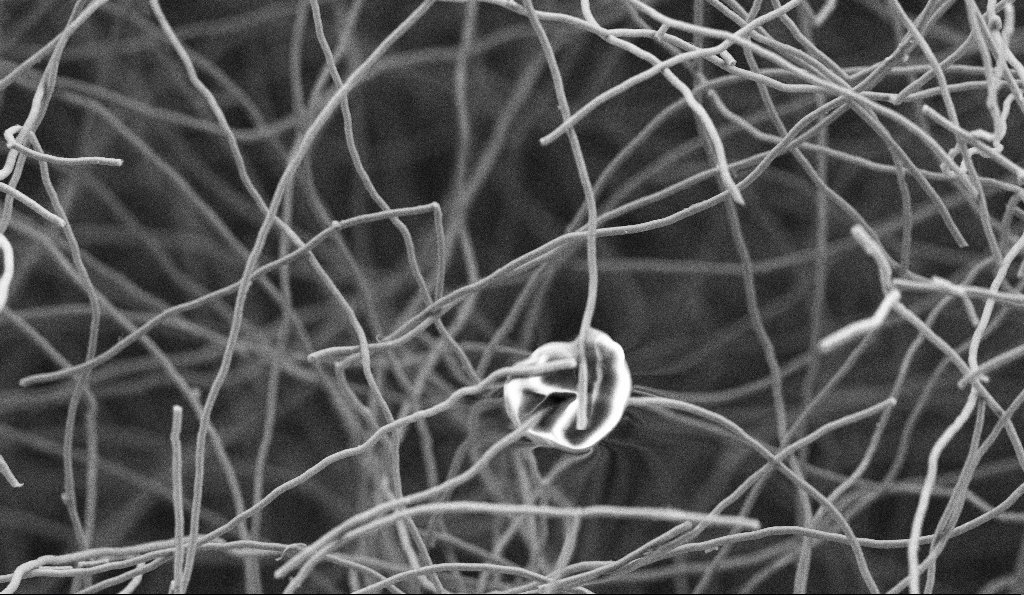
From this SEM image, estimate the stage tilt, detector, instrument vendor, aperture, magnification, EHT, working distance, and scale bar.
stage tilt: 0°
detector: SE2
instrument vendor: Zeiss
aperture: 30 µm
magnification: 5 K X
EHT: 5 kV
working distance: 4.8 mm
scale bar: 10000 nm